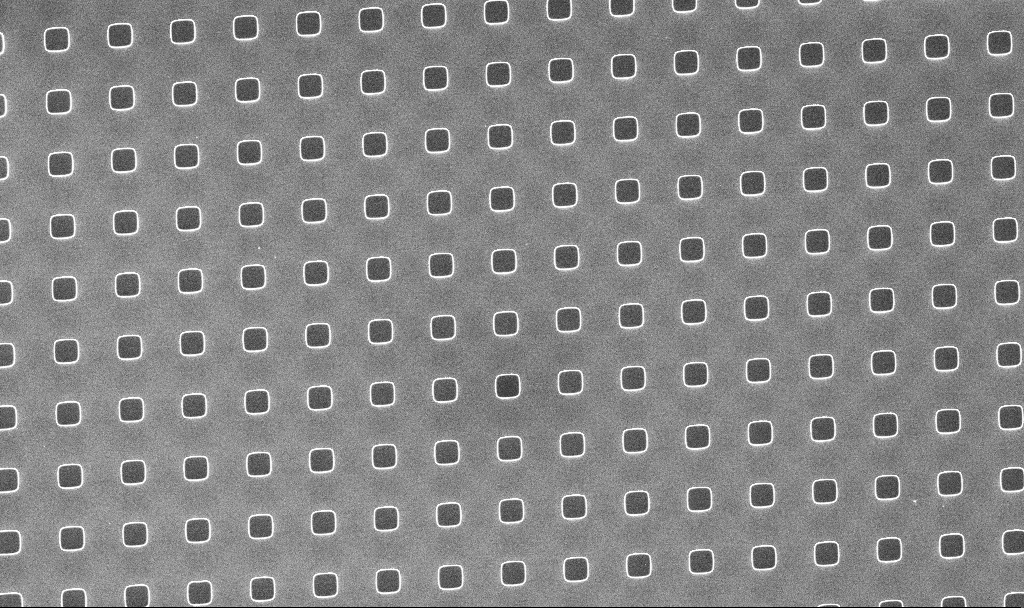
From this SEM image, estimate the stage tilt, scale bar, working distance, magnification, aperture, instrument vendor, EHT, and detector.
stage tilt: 0°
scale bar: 10000 nm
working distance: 5.1 mm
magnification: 1.93 K X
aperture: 30 µm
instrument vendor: Zeiss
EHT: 5 kV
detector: InLens